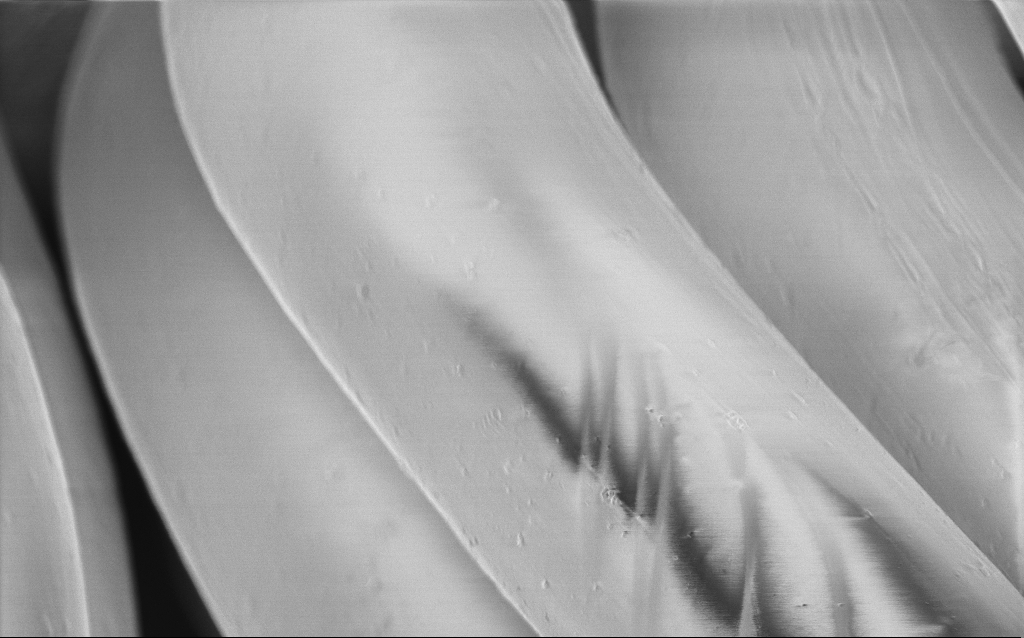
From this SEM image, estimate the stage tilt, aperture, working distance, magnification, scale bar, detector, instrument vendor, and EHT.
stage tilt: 0°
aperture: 30 µm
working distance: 5 mm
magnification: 6.24 K X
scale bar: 10000 nm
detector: InLens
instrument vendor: Zeiss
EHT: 2 kV